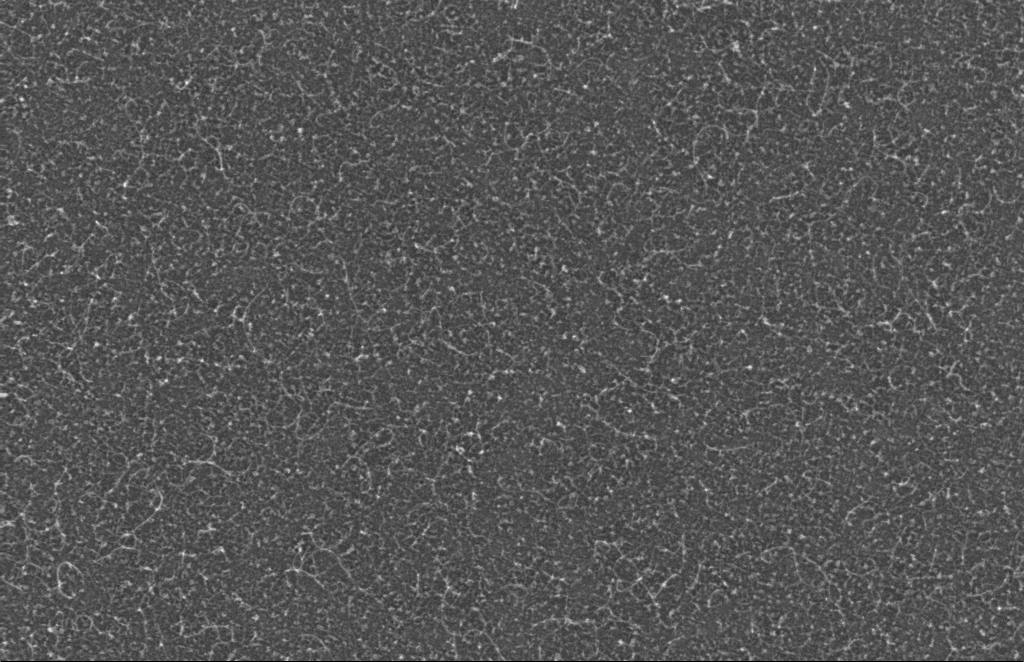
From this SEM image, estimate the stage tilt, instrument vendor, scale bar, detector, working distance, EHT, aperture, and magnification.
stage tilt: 0°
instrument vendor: Zeiss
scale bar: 200 nm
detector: InLens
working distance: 6 mm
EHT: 5 kV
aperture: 30 µm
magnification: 135.3 K X